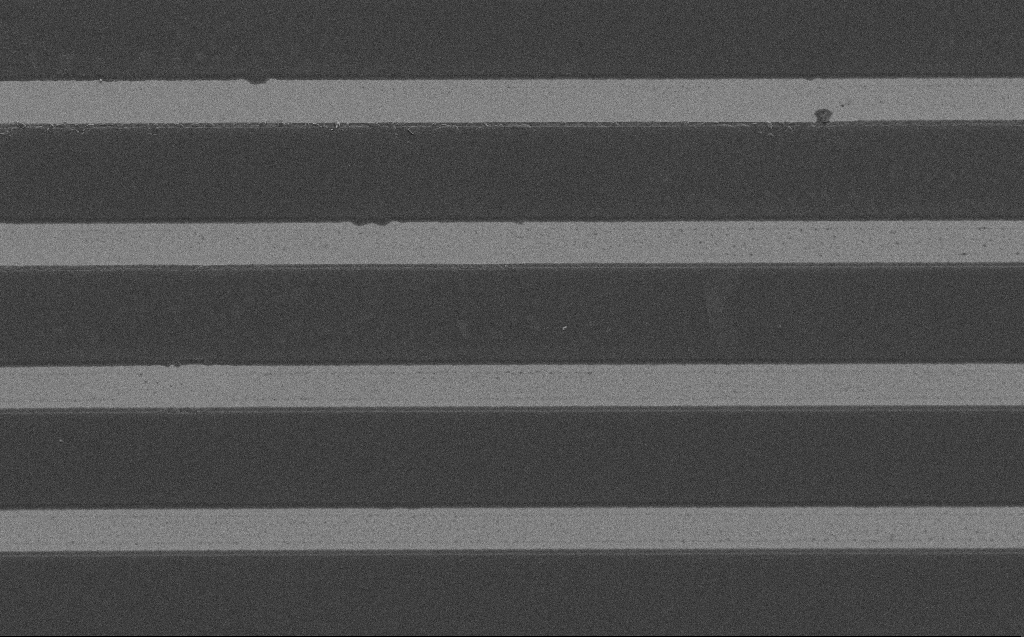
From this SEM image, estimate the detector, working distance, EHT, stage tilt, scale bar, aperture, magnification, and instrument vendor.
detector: SE2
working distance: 4 mm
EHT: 1.2 kV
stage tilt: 0°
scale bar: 20000 nm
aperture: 30 µm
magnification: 1.92 K X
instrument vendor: Zeiss